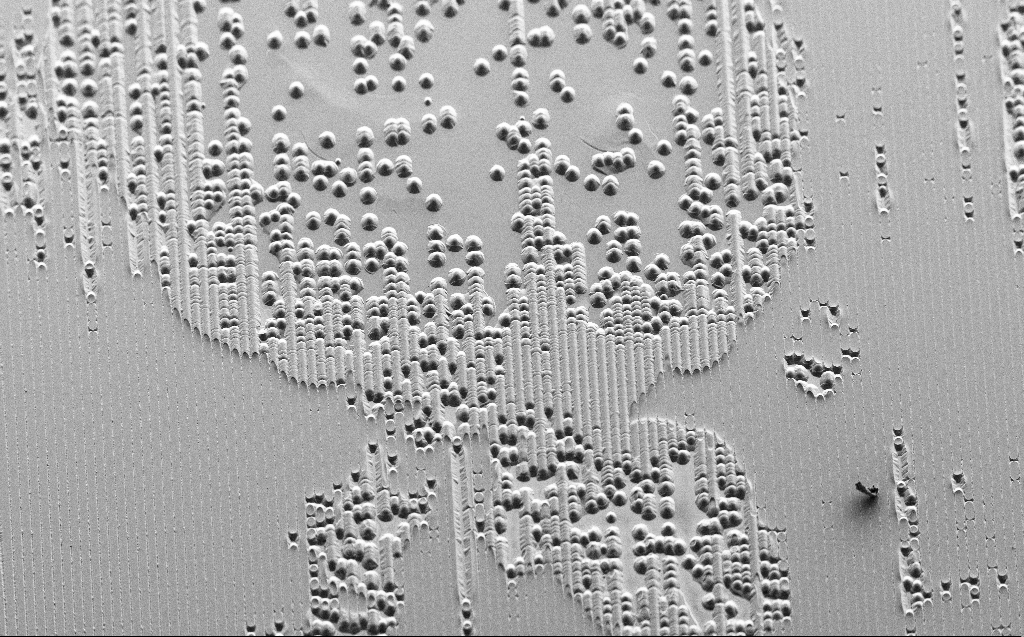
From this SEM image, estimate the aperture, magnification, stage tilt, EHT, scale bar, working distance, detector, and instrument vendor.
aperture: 30 µm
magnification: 0.344 K X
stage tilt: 45°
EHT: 3 kV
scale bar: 100000 nm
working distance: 9 mm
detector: SE2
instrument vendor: Zeiss